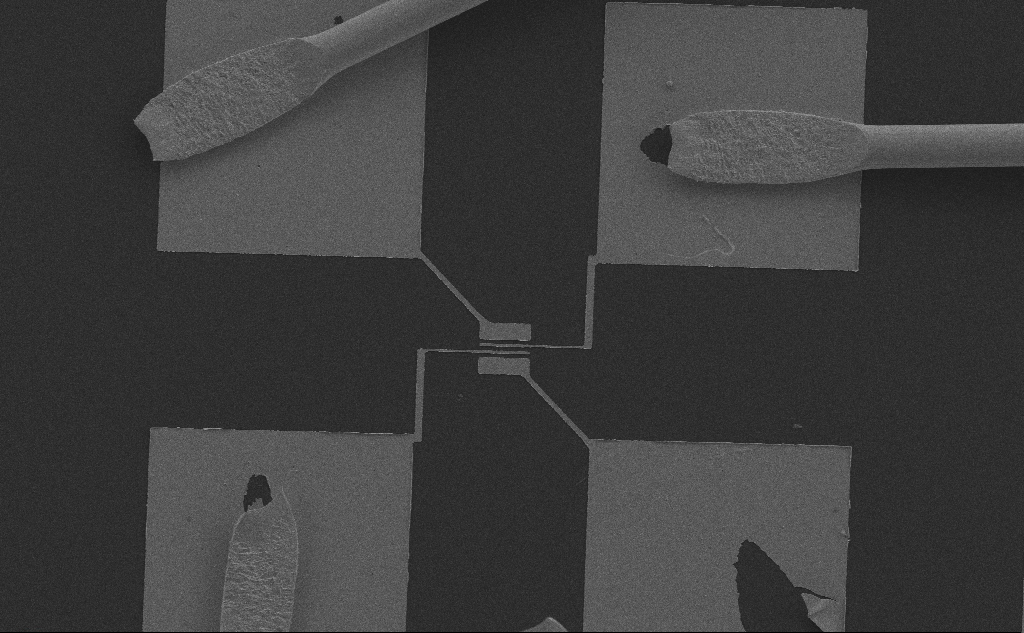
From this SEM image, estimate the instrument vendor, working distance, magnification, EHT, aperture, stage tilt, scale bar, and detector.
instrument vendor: Zeiss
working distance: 6 mm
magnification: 0.65 K X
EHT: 10 kV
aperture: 30 µm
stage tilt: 0°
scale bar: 100000 nm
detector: SE2